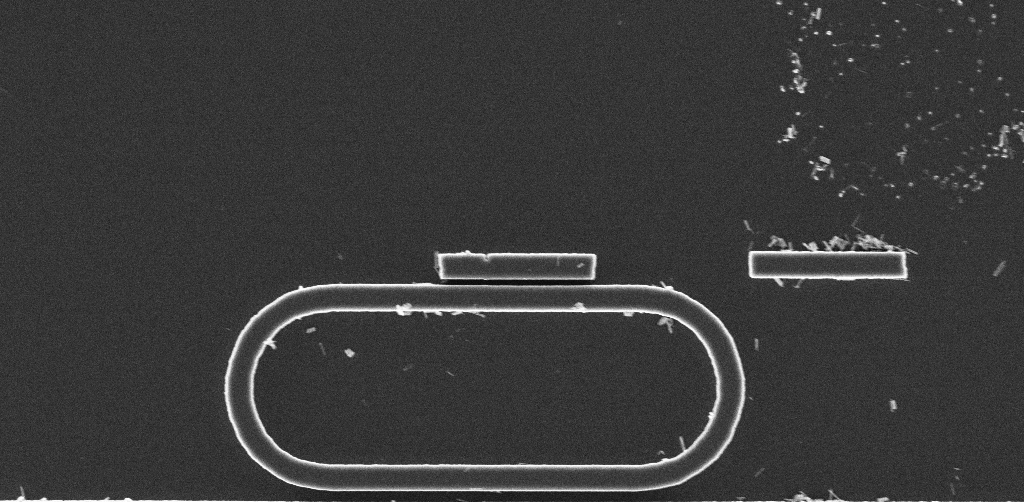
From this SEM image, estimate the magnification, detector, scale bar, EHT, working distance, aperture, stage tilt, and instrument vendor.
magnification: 19.25 K X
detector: InLens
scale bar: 2000 nm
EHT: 3 kV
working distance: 2.9 mm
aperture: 30 µm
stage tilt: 0°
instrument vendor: Zeiss